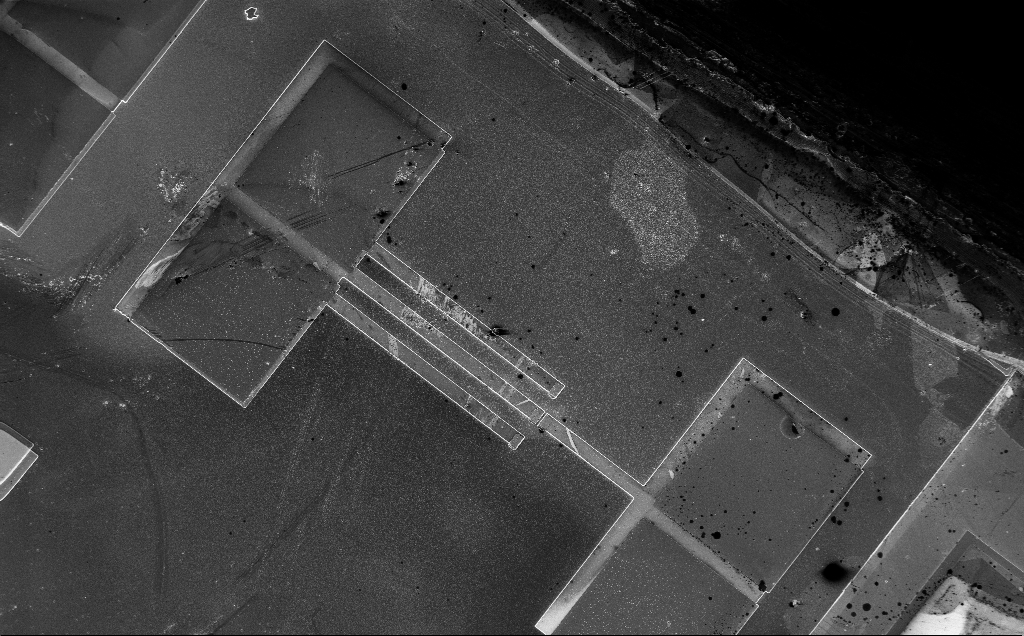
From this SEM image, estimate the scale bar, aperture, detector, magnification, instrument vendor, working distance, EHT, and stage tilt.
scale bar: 100000 nm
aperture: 30 µm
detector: InLens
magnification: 0.3 K X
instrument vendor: Zeiss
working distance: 10 mm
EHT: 5 kV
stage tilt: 0°